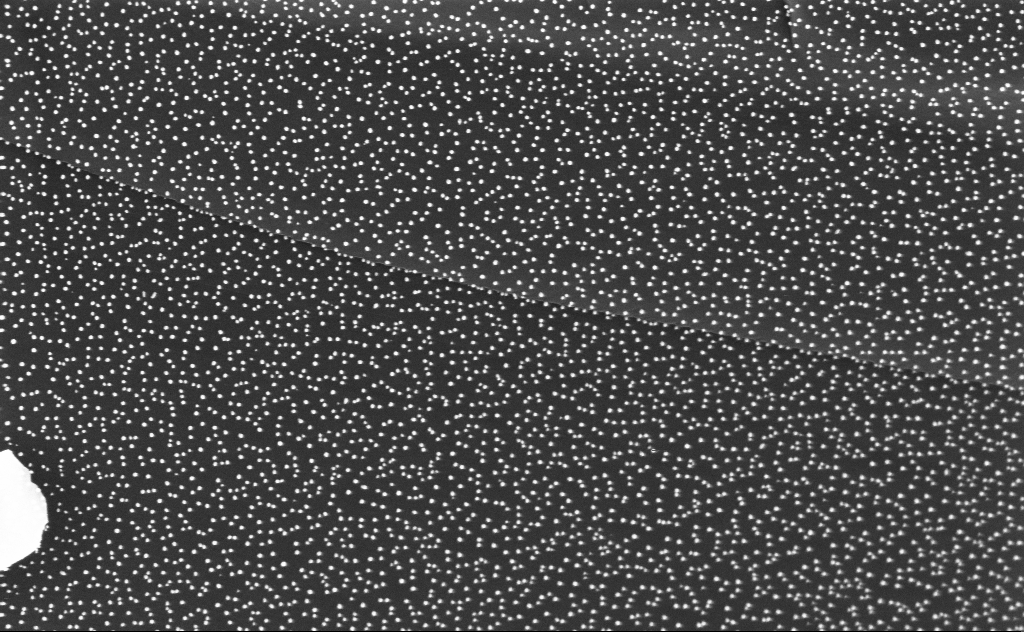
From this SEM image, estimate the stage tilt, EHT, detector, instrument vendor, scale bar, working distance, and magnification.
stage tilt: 0°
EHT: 3 kV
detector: InLens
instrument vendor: Zeiss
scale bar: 200 nm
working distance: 5 mm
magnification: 80 K X